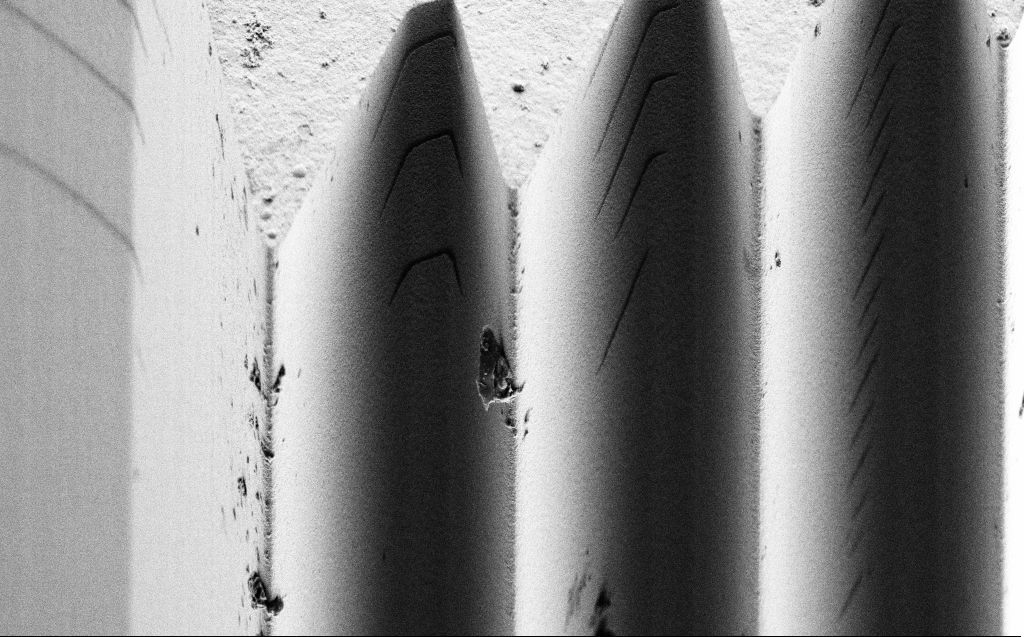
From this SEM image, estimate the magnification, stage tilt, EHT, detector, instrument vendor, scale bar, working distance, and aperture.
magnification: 8.1 K X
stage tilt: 45°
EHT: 2 kV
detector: SE2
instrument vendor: Zeiss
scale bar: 2000 nm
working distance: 6 mm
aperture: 30 µm